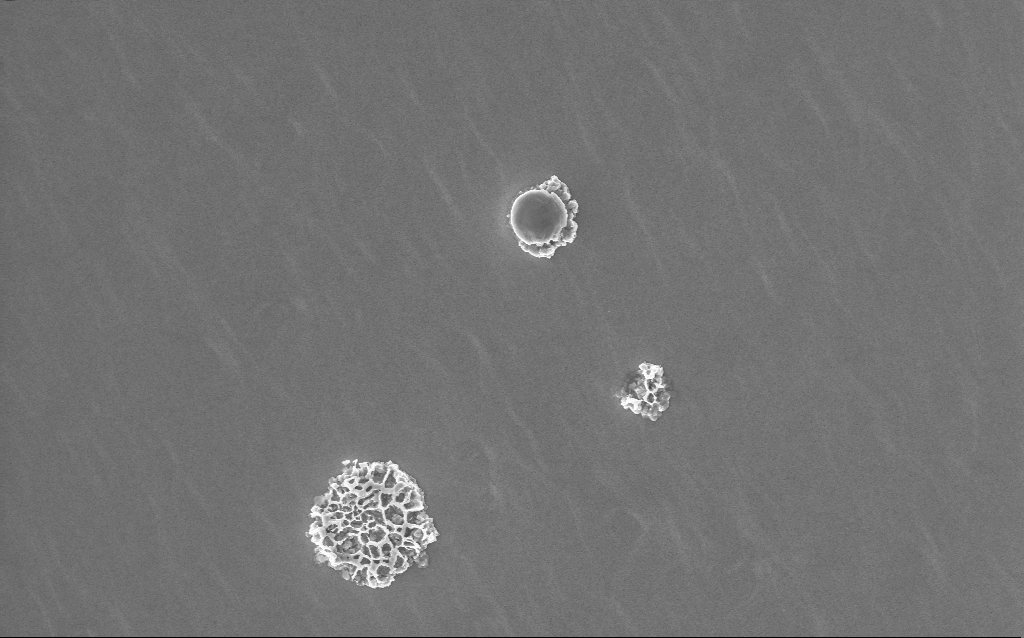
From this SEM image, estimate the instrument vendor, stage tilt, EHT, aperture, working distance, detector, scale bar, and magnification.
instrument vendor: Zeiss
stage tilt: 0°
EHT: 10 kV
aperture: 30 µm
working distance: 2 mm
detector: InLens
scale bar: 2000 nm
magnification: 13.31 K X